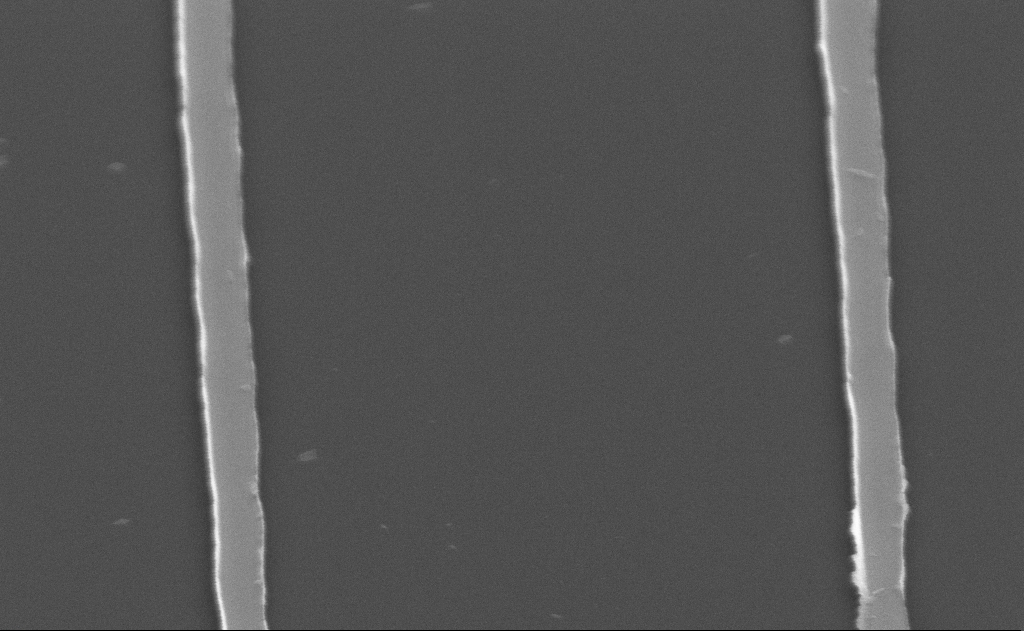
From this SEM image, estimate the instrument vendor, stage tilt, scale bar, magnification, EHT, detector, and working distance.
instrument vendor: Zeiss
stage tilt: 45°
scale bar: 1000 nm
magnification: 58.55 K X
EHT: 5 kV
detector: SE2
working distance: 12 mm